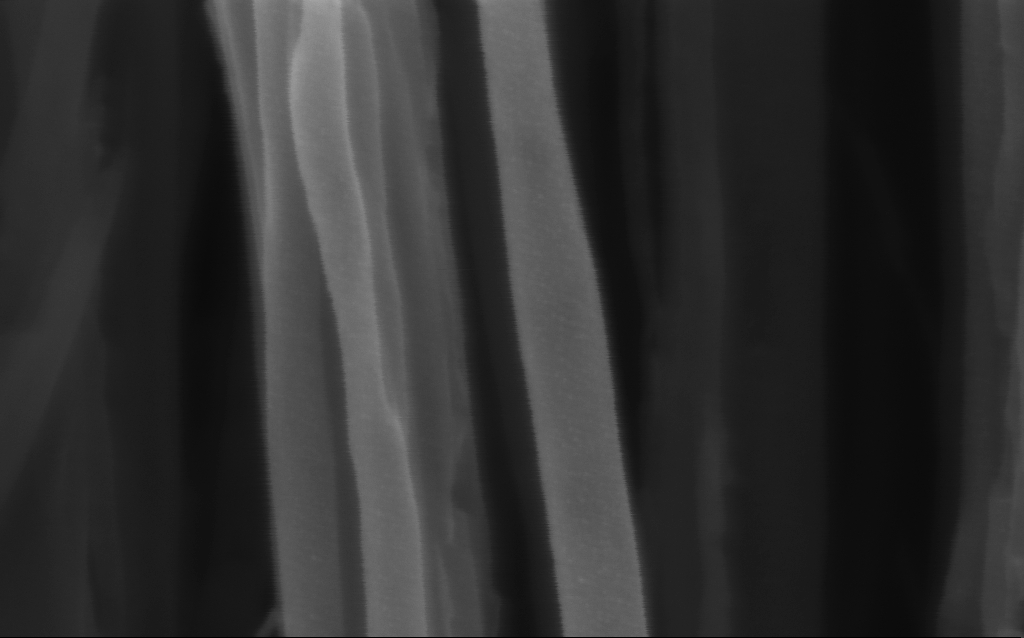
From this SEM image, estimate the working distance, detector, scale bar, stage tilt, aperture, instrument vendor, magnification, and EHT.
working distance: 3 mm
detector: InLens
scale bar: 100 nm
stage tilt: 0°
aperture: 30 µm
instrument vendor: Zeiss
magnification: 343.95 K X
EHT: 3 kV